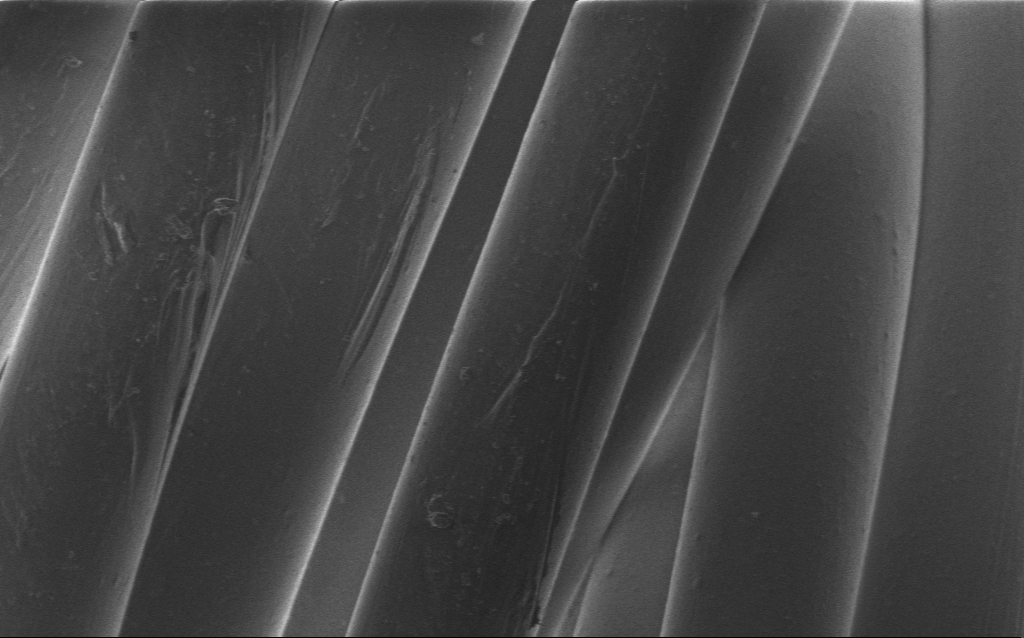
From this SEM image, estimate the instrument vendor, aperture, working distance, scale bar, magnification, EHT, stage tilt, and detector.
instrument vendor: Zeiss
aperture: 30 µm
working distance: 4 mm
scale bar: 20000 nm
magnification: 2.81 K X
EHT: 1 kV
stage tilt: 0°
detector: InLens